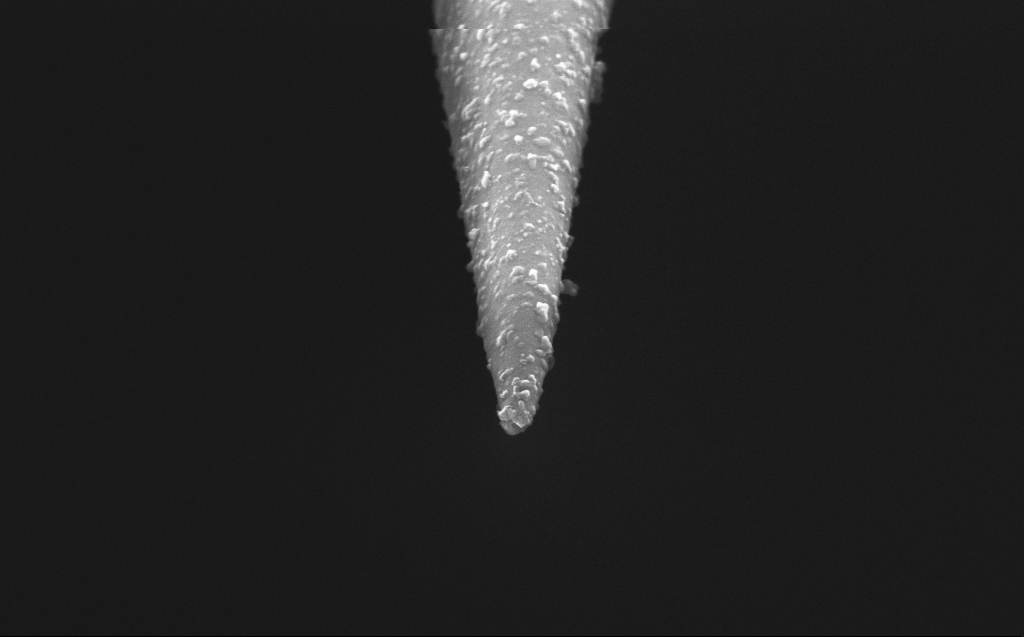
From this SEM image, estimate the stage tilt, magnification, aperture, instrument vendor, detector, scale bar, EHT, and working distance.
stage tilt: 45°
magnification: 100 K X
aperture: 30 µm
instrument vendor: Zeiss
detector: InLens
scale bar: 200 nm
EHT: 5 kV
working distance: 3 mm